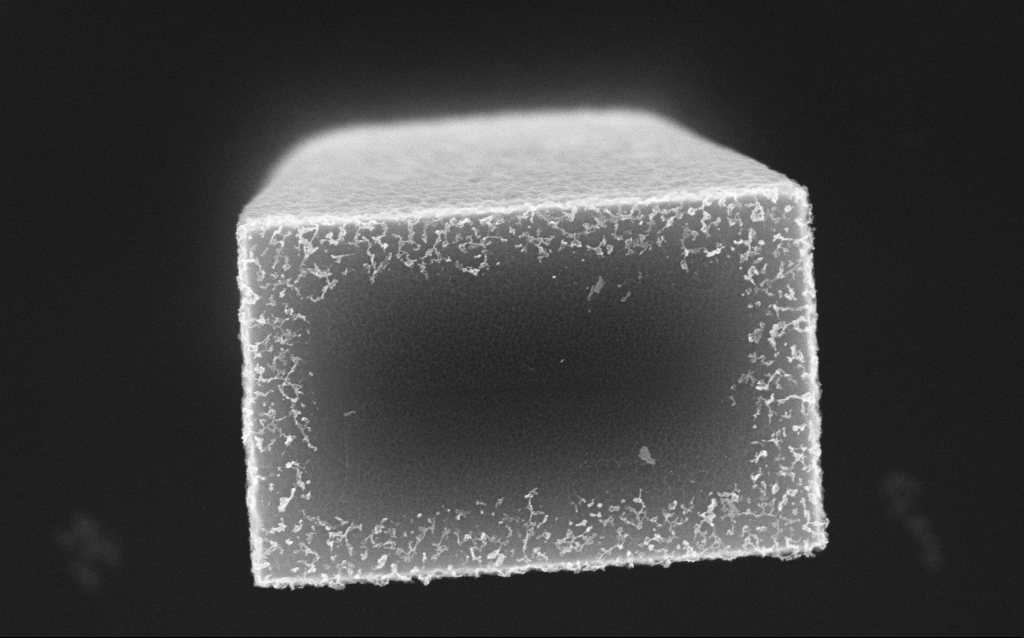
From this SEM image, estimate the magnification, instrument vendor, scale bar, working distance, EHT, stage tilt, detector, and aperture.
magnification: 72.97 K X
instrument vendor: Zeiss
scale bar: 200 nm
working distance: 8.4 mm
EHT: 10 kV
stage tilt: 0°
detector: InLens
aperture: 30 µm